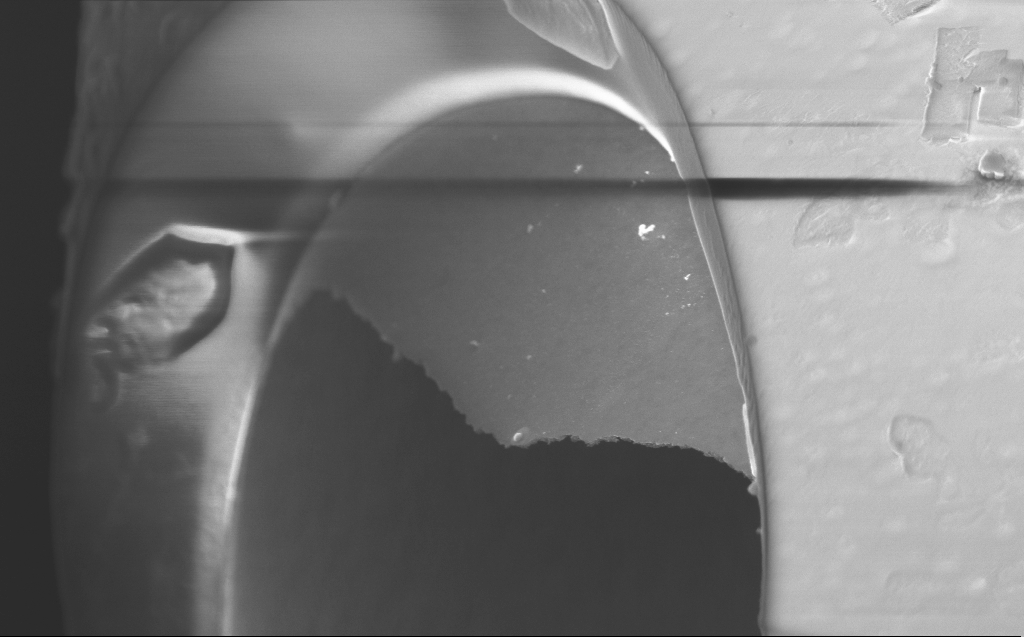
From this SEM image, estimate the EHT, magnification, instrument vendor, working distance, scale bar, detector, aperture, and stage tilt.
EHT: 5 kV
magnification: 32.81 K X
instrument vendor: Zeiss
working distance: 4 mm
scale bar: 1000 nm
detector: InLens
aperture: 30 µm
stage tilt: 45°